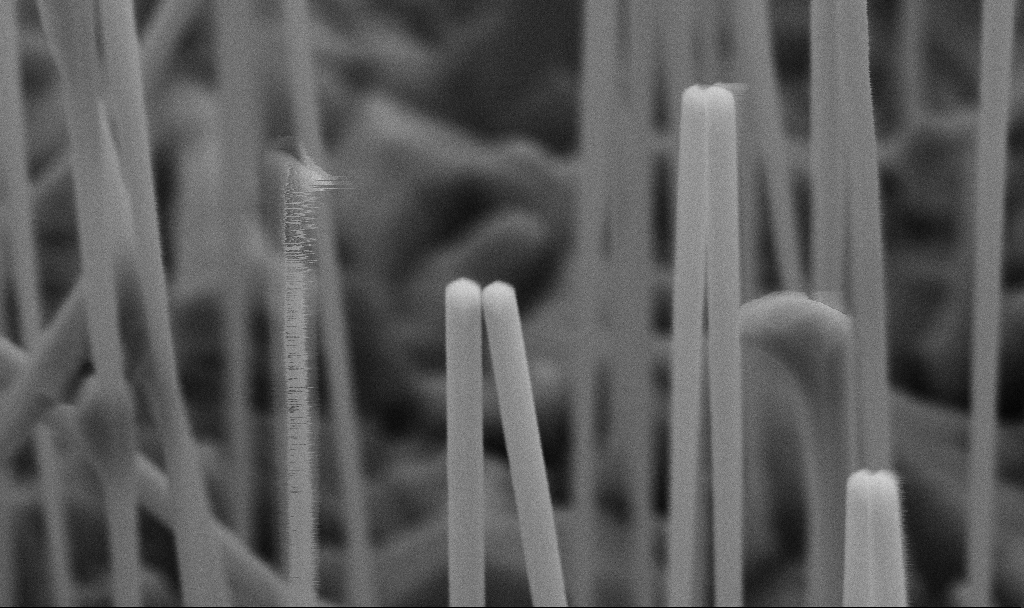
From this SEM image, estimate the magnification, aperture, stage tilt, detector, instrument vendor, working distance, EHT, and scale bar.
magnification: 100 K X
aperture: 30 µm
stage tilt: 45°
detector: SE2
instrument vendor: Zeiss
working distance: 7.3 mm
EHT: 5 kV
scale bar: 200 nm